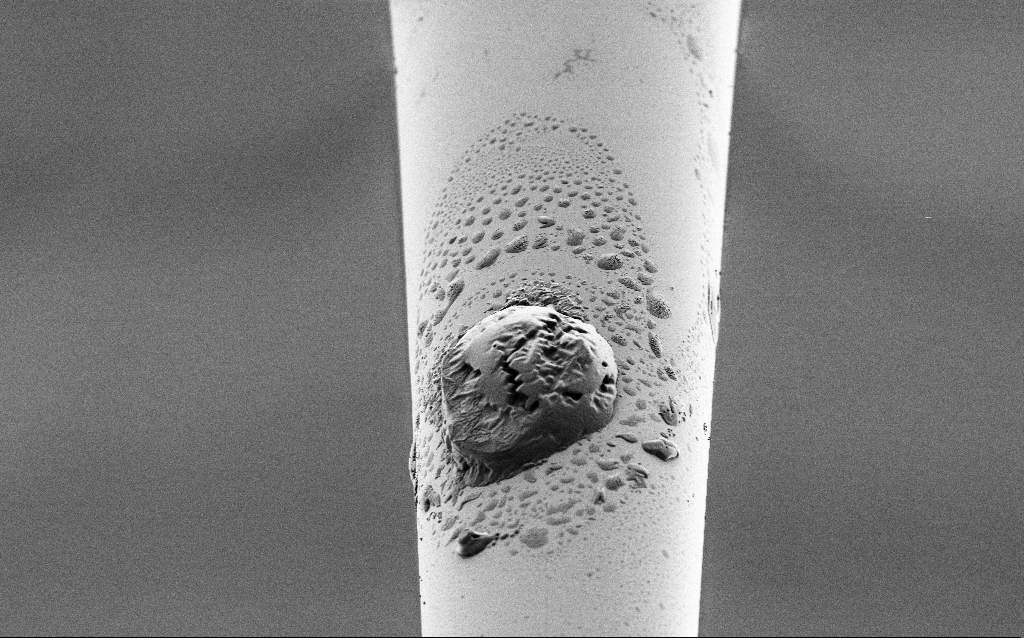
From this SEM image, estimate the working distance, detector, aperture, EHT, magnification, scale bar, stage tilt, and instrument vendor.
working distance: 6.5 mm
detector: SE2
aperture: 30 µm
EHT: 1 kV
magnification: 1.5 K X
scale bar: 10000 nm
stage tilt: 45°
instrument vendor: Zeiss